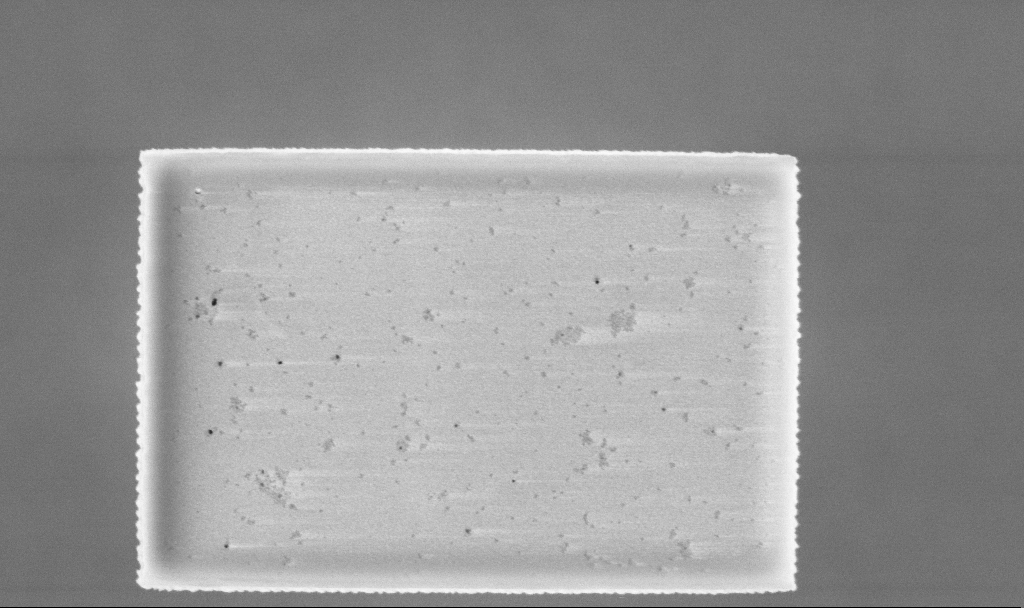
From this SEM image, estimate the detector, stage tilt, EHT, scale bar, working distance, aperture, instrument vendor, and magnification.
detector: InLens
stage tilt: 0°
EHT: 5 kV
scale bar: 1000 nm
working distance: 3.2 mm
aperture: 30 µm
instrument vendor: Zeiss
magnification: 52.84 K X